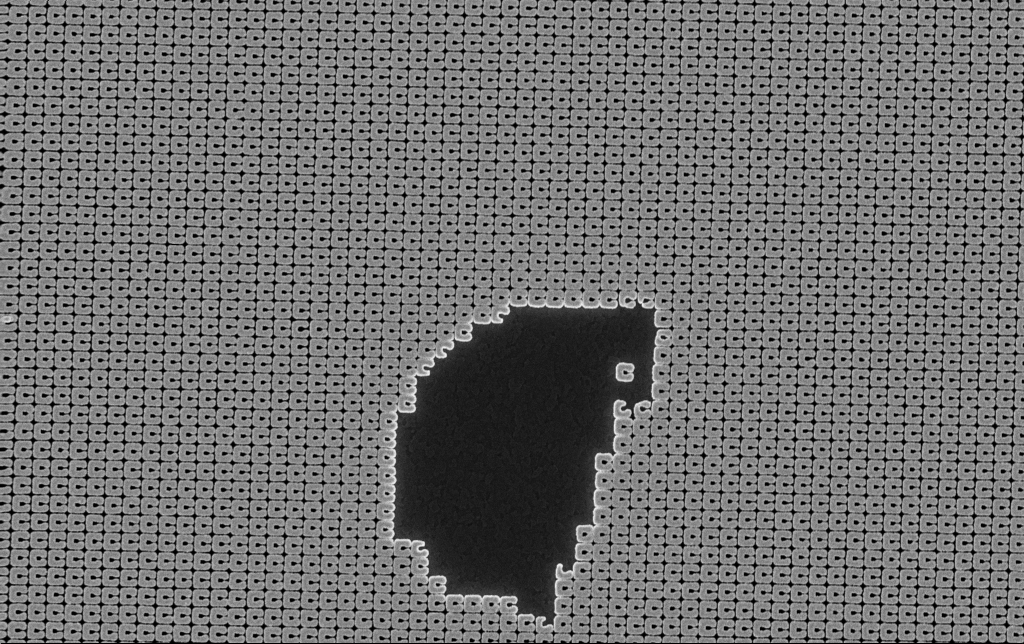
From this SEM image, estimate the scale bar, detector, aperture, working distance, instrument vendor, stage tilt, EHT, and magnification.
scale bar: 2000 nm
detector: InLens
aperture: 30 µm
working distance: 3.9 mm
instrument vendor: Zeiss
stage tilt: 0°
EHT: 5 kV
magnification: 14.53 K X